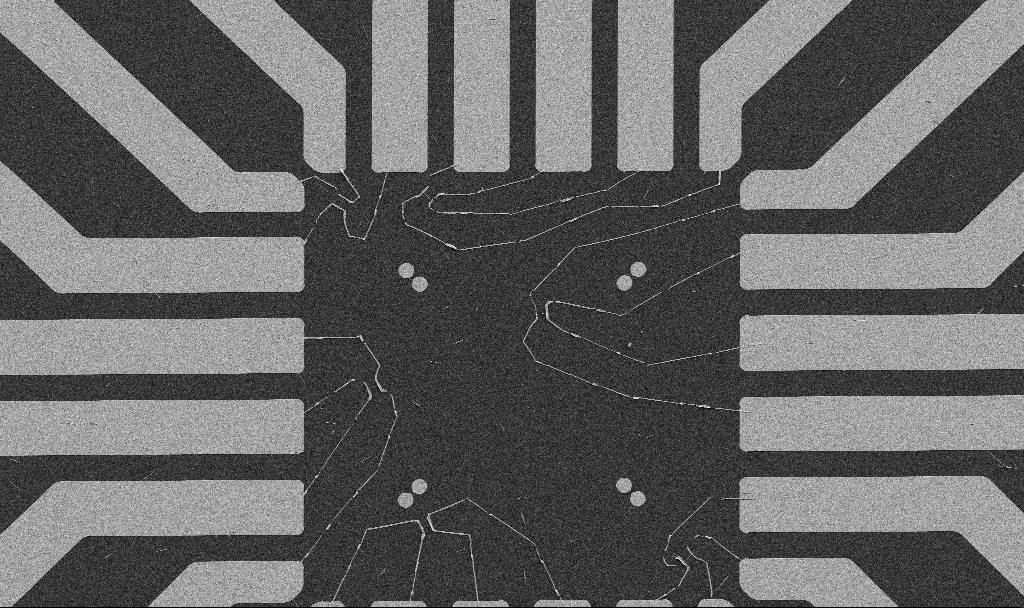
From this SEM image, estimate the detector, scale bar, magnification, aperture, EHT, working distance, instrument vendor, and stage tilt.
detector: SE2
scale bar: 20000 nm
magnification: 1 K X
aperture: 30 µm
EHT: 5 kV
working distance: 10.7 mm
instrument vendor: Zeiss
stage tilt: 0°